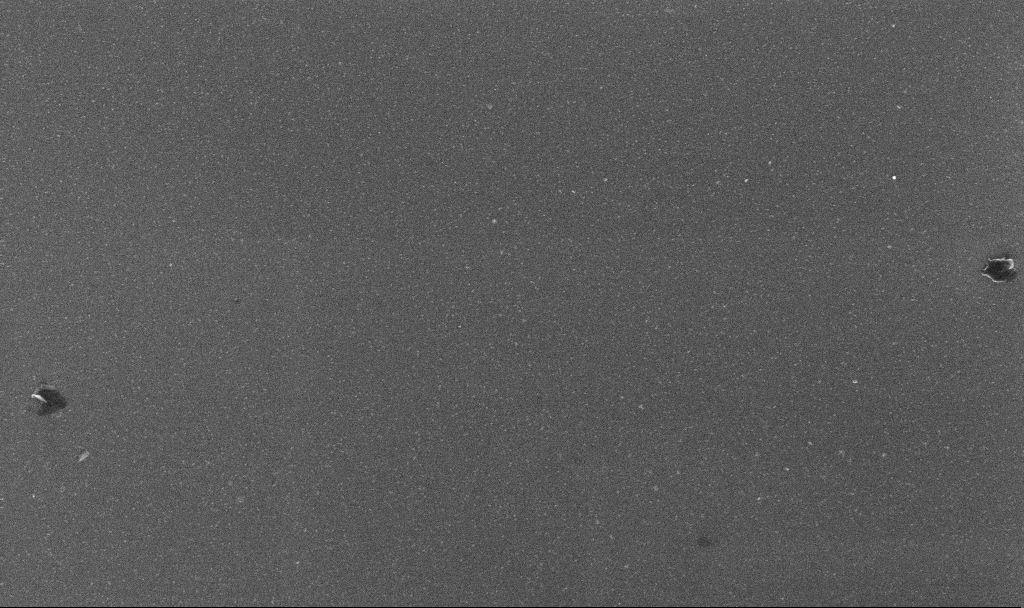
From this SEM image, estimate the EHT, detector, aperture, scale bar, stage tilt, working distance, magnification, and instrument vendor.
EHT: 10 kV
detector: InLens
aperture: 30 µm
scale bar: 1000 nm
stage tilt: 0°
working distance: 3.2 mm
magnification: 50 K X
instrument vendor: Zeiss